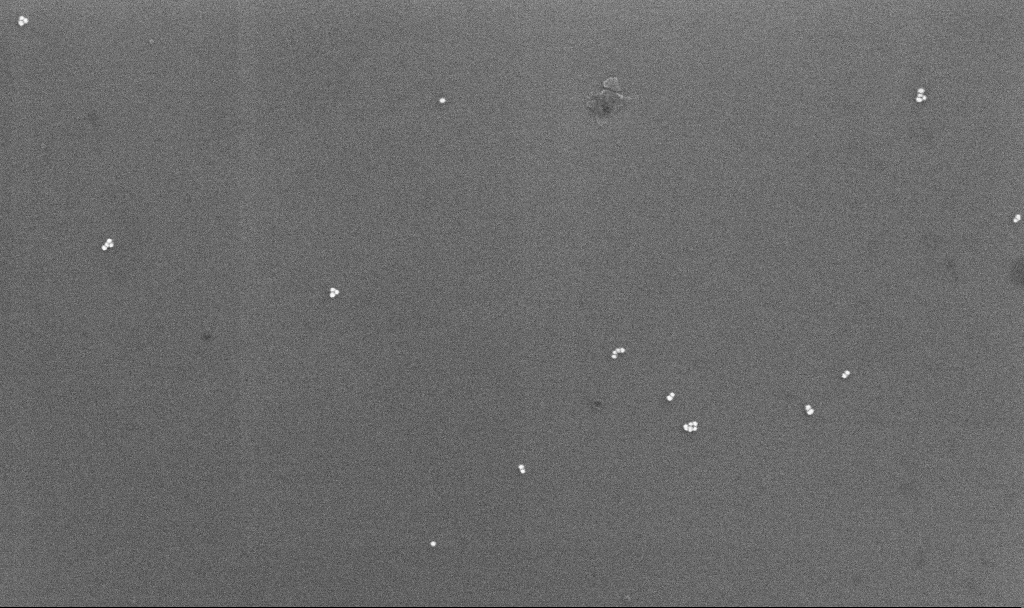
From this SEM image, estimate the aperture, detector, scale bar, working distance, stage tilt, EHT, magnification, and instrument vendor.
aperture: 30 µm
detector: InLens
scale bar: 200 nm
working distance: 4.3 mm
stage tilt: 0°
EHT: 10 kV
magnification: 100 K X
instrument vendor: Zeiss